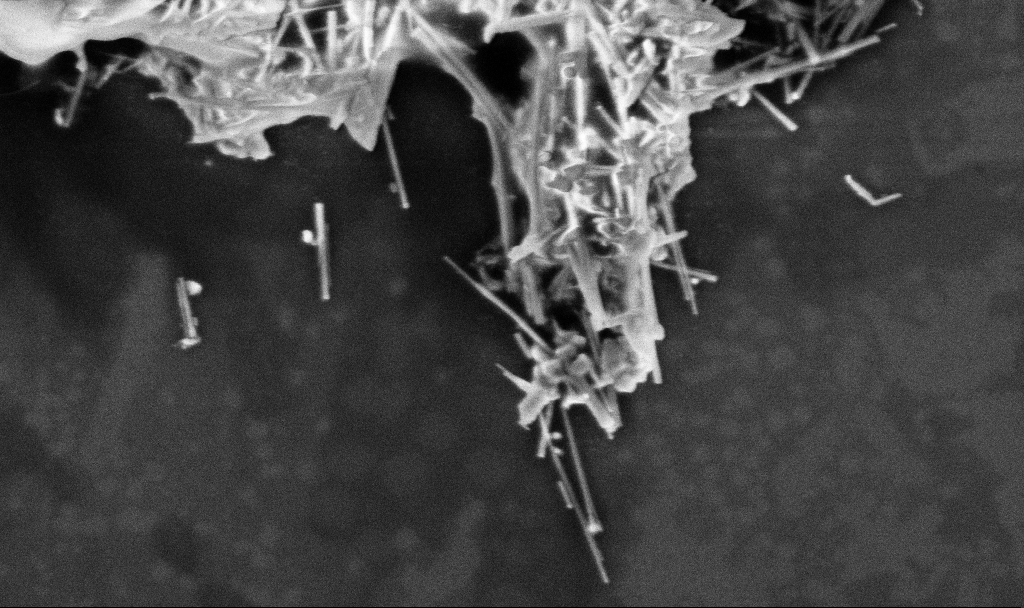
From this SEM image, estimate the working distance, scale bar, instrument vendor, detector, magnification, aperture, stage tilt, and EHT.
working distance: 3.3 mm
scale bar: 1000 nm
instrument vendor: Zeiss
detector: InLens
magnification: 58.78 K X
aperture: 30 µm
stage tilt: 0°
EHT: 3 kV